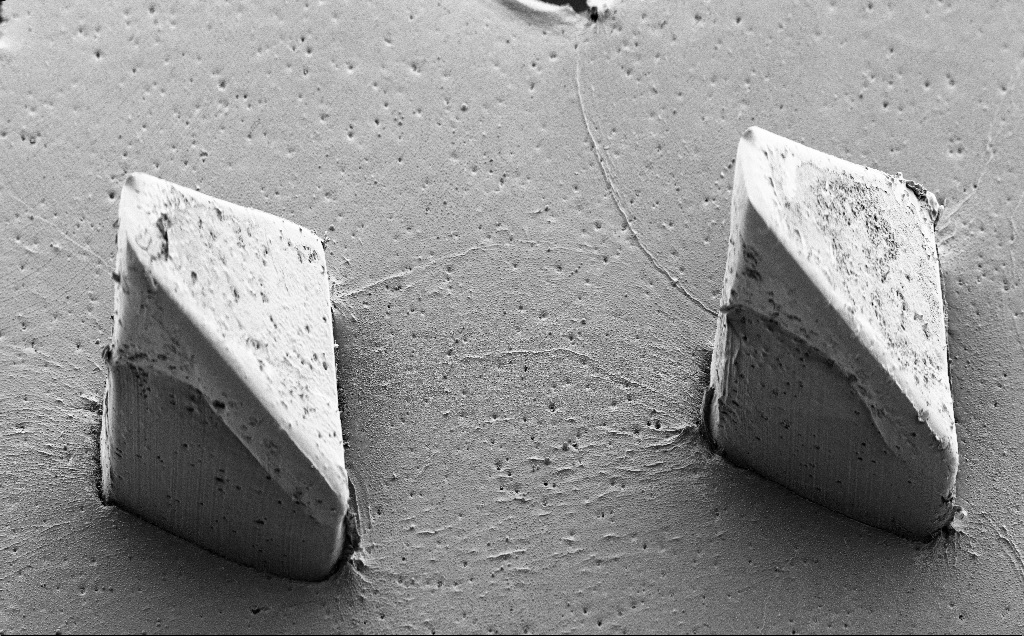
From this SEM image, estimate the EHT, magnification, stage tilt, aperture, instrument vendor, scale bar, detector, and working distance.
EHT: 5 kV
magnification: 0.241 K X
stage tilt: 40°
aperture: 30 µm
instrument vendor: Zeiss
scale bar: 100000 nm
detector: SE2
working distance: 9 mm